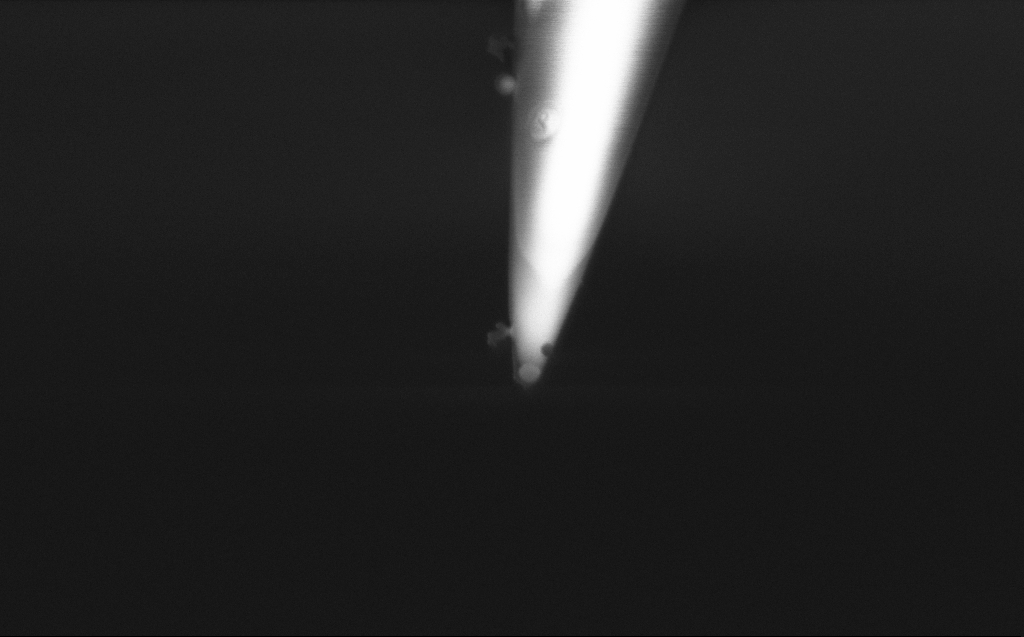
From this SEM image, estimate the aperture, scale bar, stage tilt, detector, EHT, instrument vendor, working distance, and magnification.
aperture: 30 µm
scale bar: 200 nm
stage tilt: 45.1°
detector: InLens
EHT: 2 kV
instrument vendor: Zeiss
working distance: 5 mm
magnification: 74.54 K X